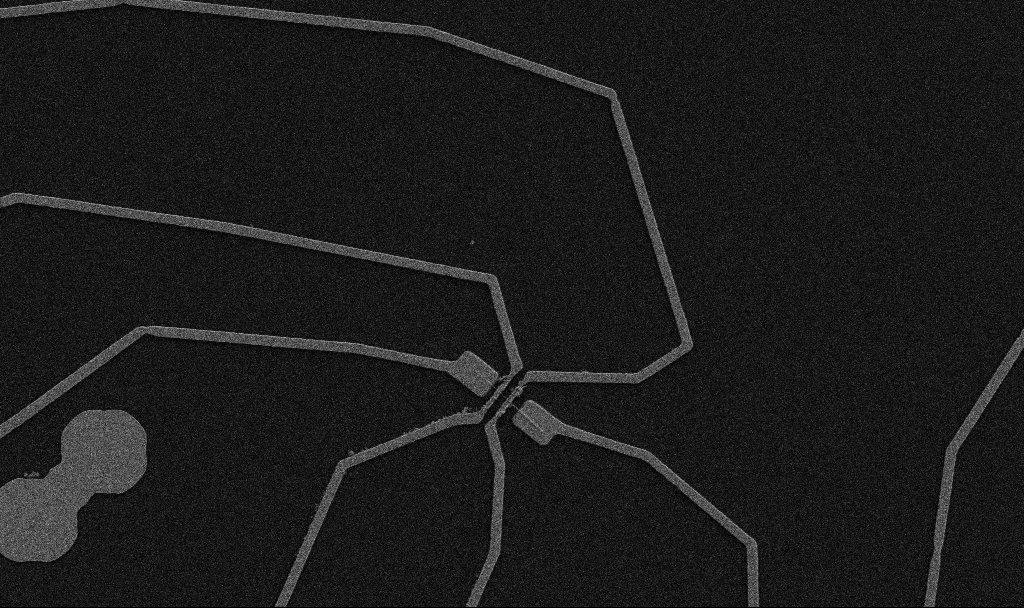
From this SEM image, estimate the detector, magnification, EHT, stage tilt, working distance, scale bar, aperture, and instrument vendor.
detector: SE2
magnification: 5 K X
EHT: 5 kV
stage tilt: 0°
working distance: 10.7 mm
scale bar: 10000 nm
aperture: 30 µm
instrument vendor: Zeiss